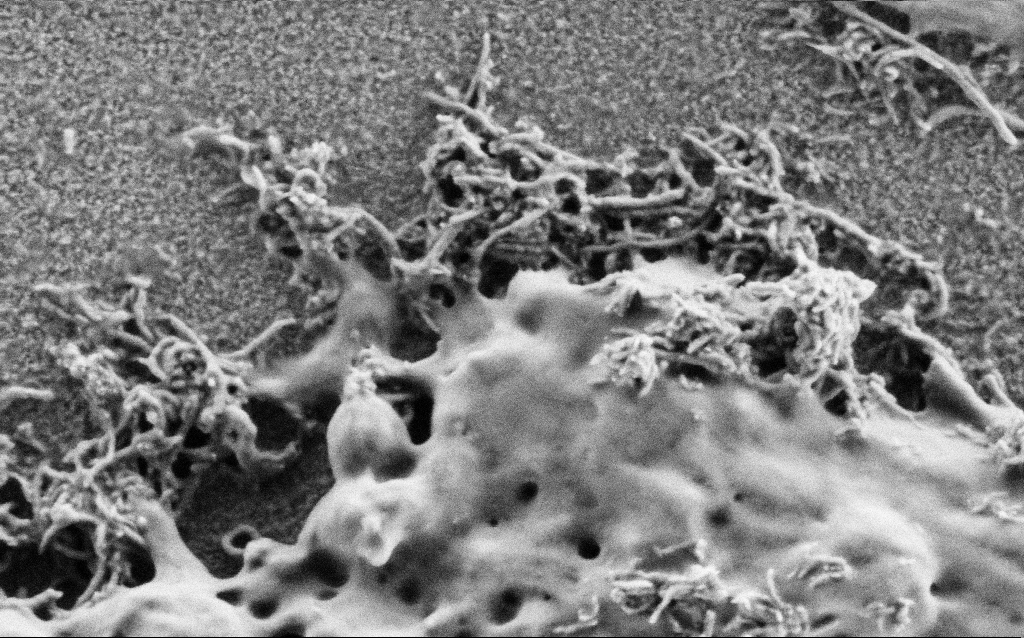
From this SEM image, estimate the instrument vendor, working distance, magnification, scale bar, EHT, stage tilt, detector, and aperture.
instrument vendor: Zeiss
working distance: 4 mm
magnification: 100 K X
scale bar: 200 nm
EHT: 1 kV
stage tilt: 0°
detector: SE2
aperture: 30 µm